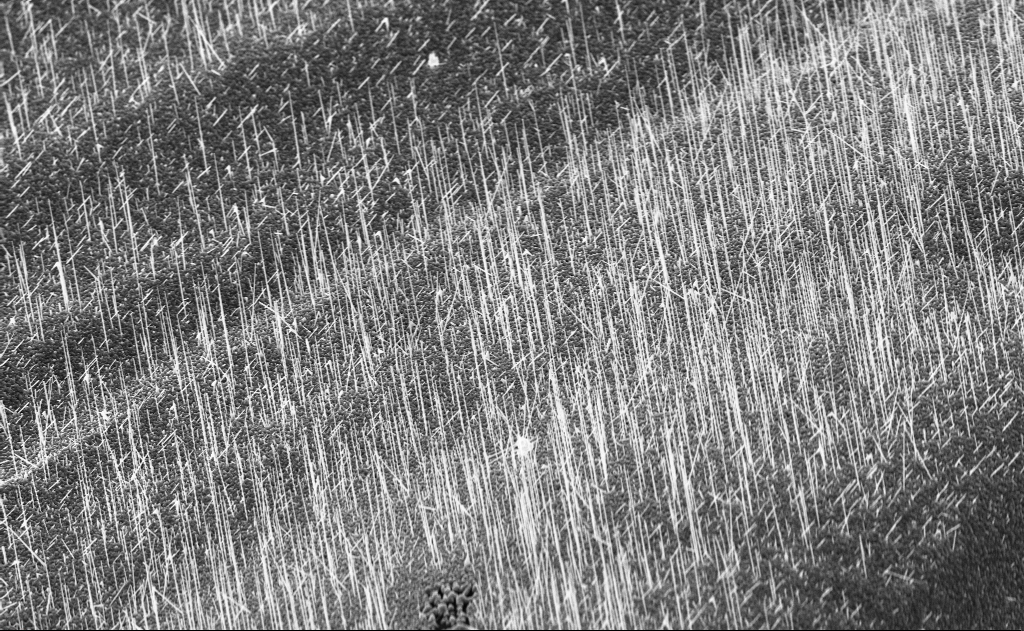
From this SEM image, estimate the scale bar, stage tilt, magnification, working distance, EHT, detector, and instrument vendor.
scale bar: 10000 nm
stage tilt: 0°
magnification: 5 K X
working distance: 8 mm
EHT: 10 kV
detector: InLens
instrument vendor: Zeiss